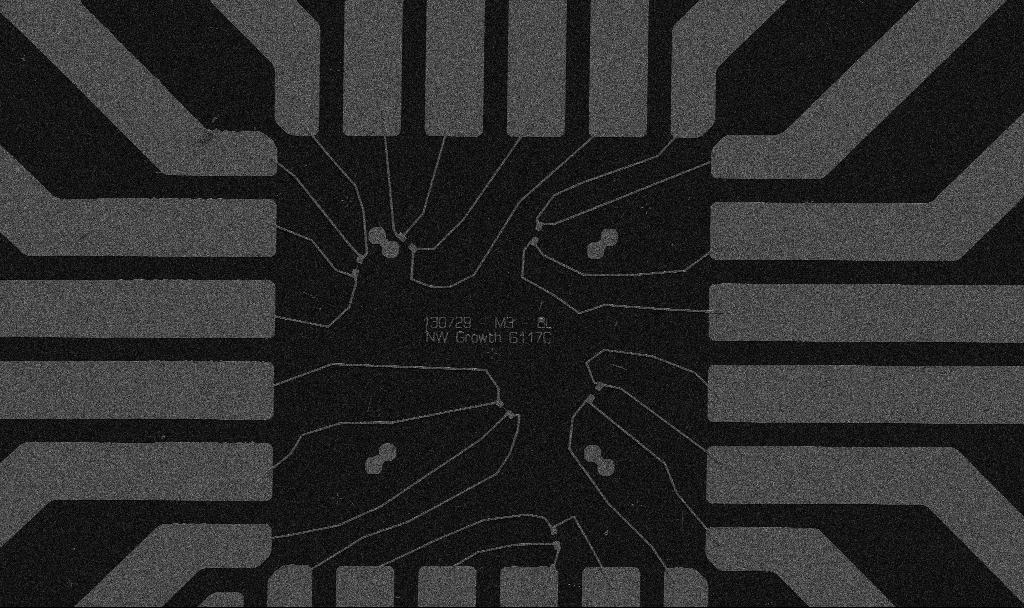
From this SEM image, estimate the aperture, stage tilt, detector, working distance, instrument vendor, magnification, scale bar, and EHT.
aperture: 30 µm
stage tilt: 0°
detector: SE2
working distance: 10.7 mm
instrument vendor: Zeiss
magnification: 1 K X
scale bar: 20000 nm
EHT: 5 kV